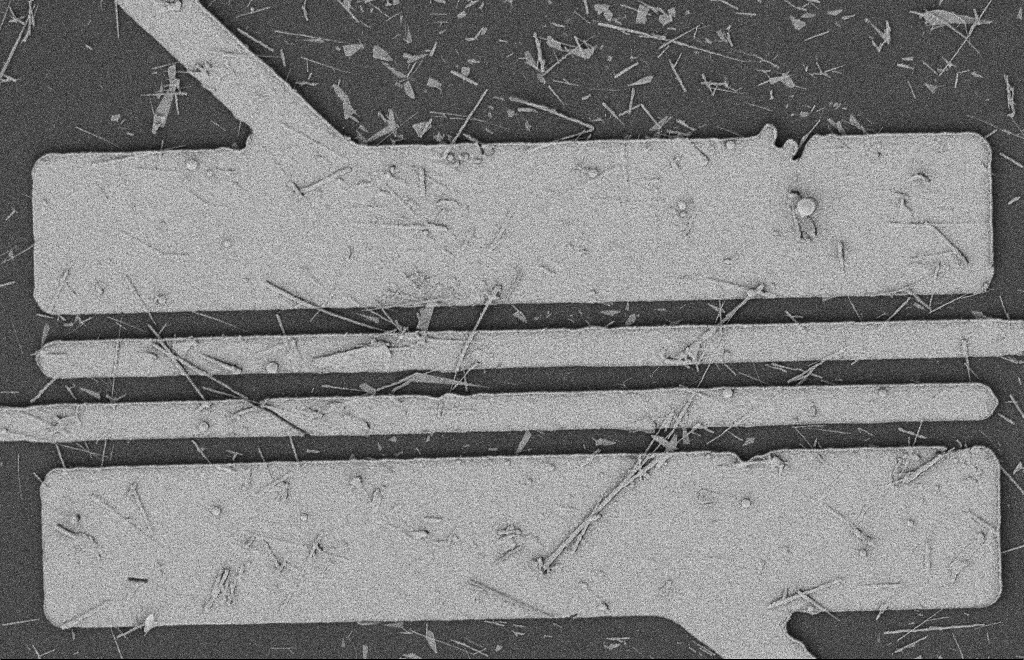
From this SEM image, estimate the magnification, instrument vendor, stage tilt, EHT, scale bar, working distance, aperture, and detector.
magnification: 5.73 K X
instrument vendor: Zeiss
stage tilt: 0°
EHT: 2 kV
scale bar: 2000 nm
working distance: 12 mm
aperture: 20 µm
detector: SE2